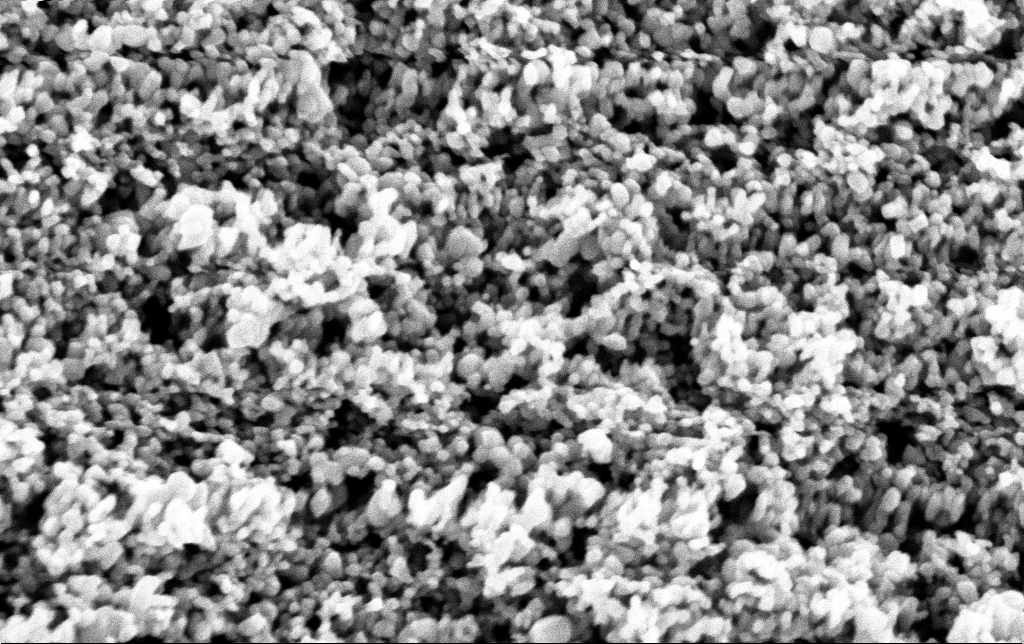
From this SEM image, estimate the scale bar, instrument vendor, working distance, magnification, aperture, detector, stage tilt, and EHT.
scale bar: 200 nm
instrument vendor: Zeiss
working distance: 2.8 mm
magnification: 173.88 K X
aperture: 30 µm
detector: InLens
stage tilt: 0°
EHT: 3 kV